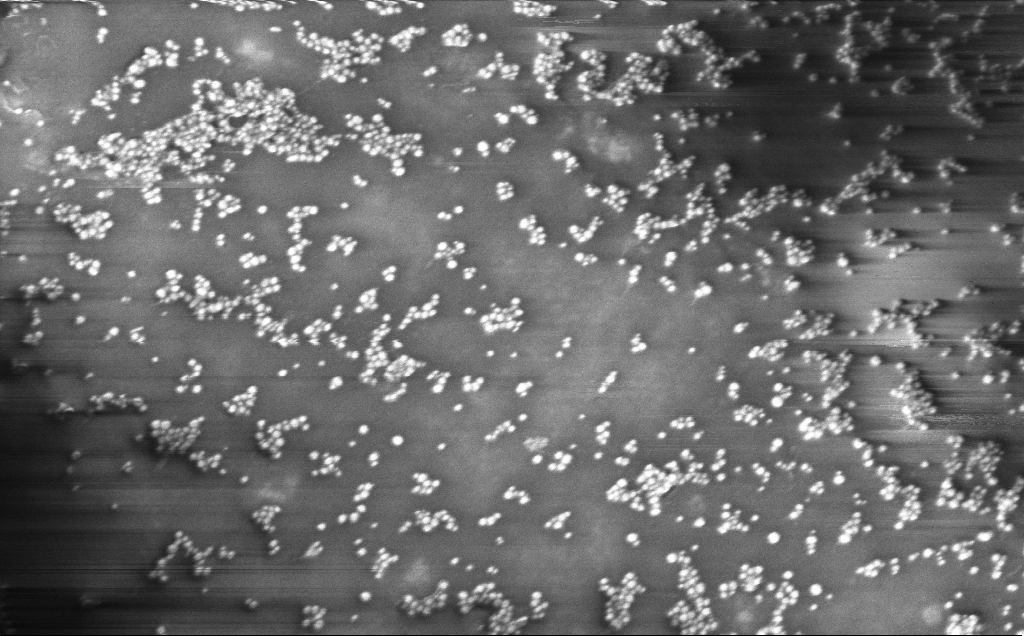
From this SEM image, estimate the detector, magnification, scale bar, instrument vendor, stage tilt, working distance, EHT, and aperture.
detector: InLens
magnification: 118.32 K X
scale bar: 200 nm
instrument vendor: Zeiss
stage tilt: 0°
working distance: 4 mm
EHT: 10 kV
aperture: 30 µm